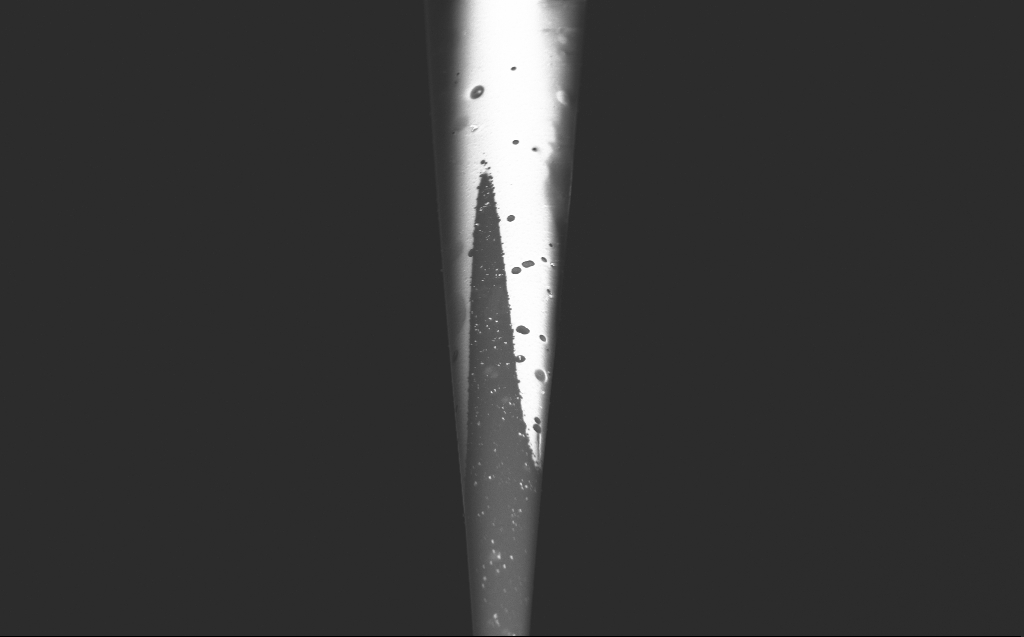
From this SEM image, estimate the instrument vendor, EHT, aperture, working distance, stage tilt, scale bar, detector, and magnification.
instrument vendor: Zeiss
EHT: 5 kV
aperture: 30 µm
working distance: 4 mm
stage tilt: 45°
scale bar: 10000 nm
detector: InLens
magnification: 4.47 K X